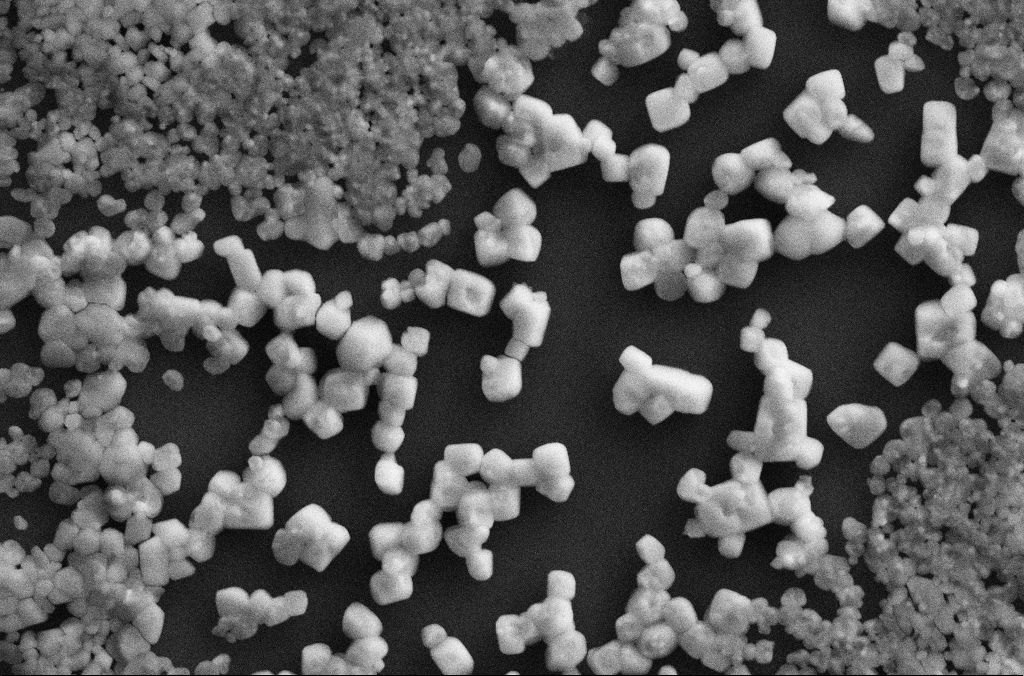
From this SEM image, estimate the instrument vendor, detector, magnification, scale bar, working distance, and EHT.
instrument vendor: Zeiss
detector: SE2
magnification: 6 K X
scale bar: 10000 nm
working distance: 2.8 mm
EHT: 20 kV